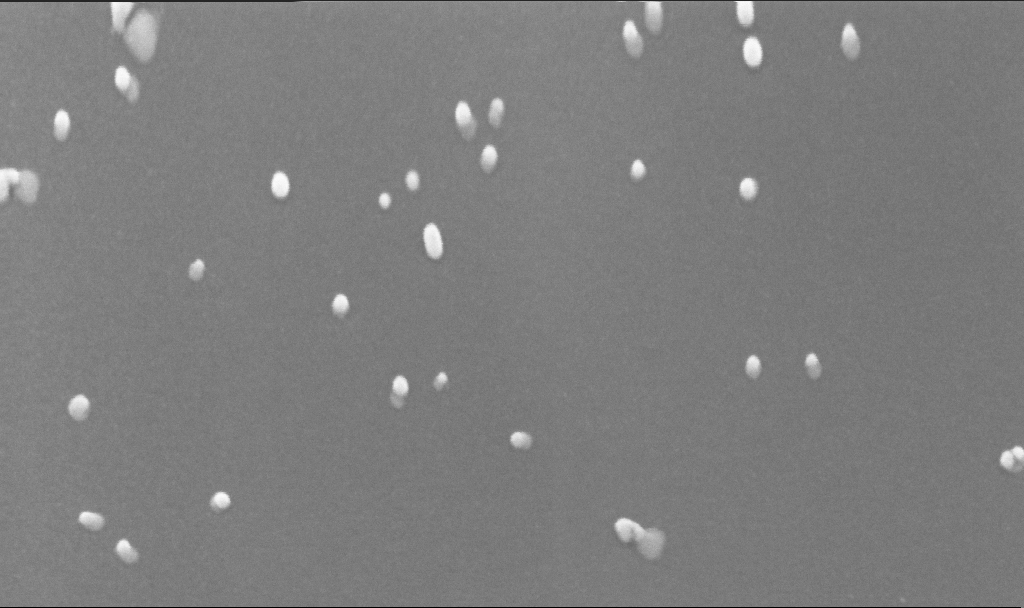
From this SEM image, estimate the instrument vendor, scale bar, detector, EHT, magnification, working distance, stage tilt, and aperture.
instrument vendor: Zeiss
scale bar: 200 nm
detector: InLens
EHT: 10 kV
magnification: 250 K X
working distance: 3.8 mm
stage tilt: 45°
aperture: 30 µm